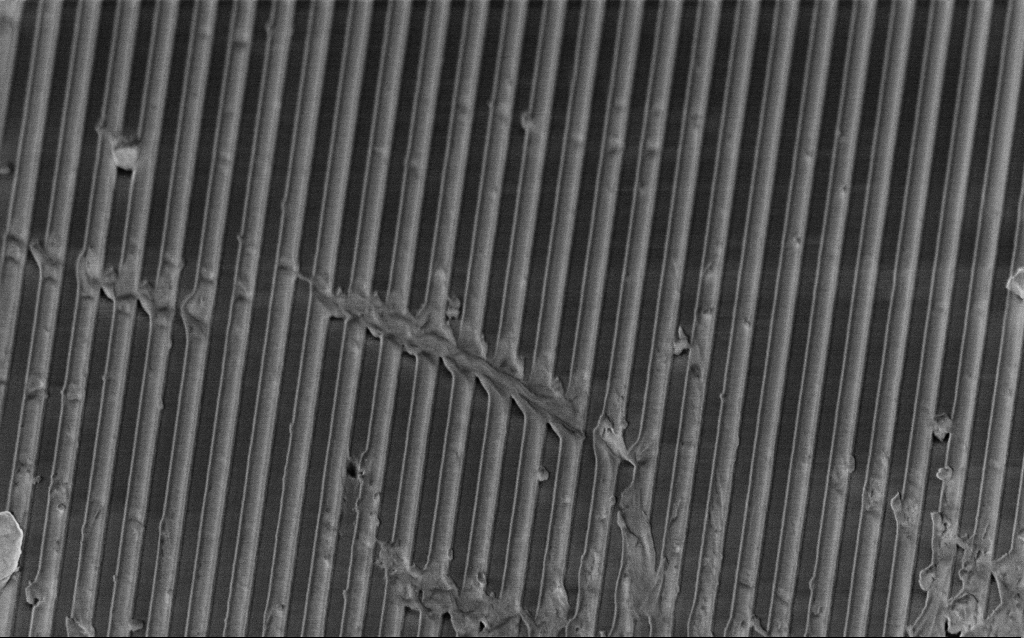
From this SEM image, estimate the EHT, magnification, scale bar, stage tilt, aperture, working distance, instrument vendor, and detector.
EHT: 2 kV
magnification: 28.46 K X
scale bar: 1000 nm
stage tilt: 45°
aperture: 30 µm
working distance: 4.1 mm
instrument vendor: Zeiss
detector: InLens